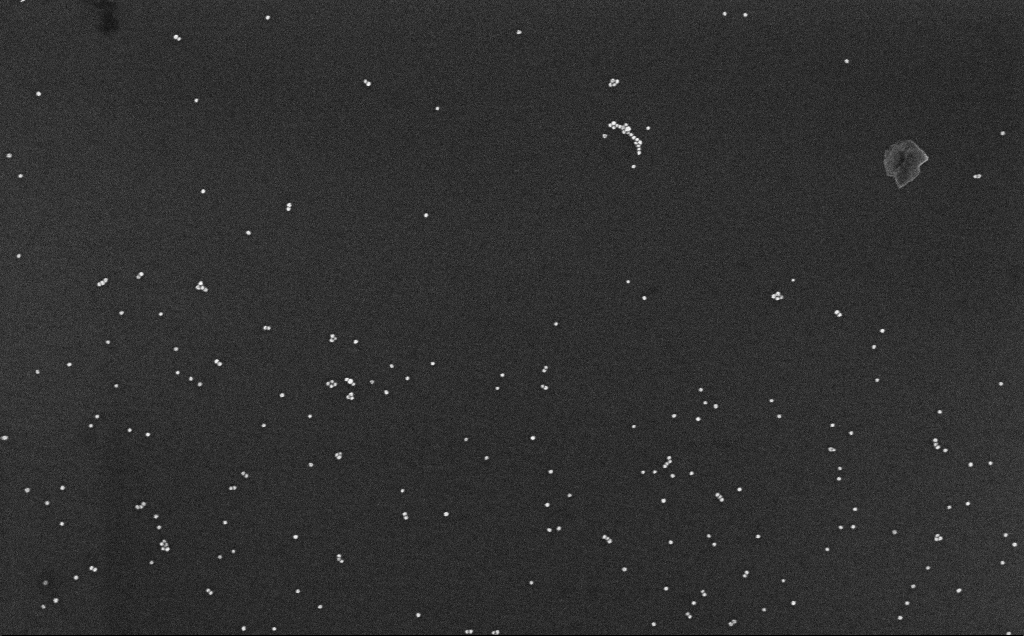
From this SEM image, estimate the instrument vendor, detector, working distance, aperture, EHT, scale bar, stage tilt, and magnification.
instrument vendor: Zeiss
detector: InLens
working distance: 6.6 mm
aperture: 30 µm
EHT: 10 kV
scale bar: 200 nm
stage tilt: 0°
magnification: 100 K X